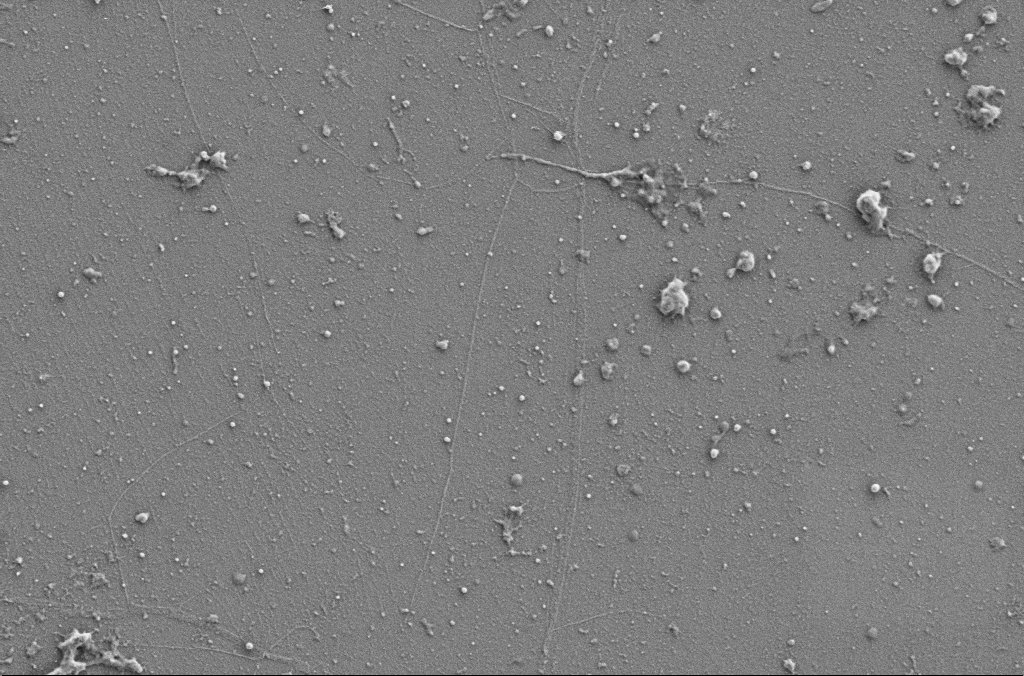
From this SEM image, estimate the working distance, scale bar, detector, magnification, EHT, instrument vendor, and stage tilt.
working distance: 4.1 mm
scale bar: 1000 nm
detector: SE2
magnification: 25 K X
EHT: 1 kV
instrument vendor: Zeiss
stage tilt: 0°